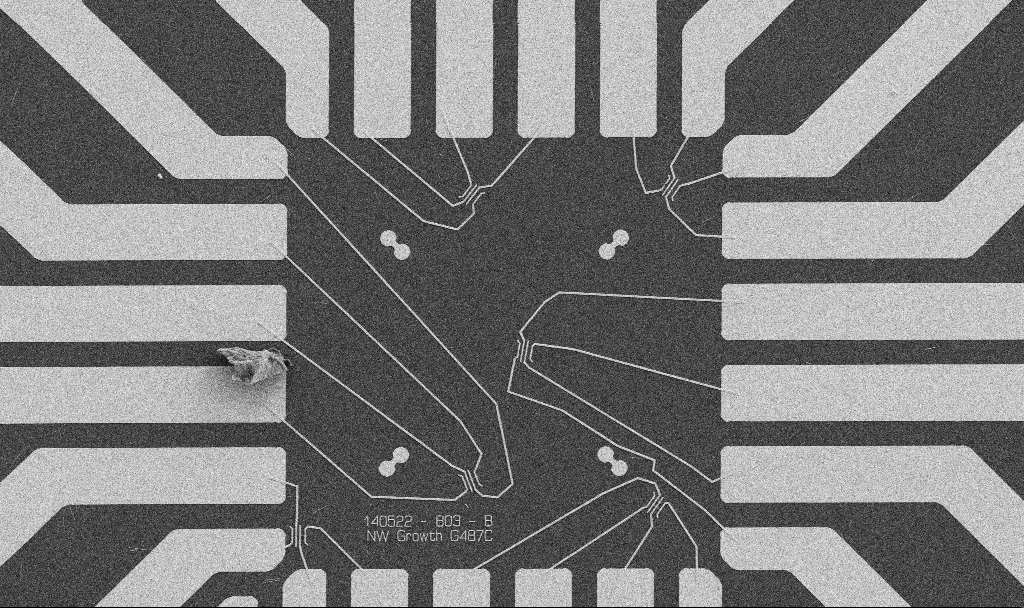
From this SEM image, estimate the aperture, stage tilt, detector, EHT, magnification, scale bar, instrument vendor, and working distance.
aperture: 30 µm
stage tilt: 0°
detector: SE2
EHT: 5 kV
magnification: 1 K X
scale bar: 20000 nm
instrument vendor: Zeiss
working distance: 10.7 mm